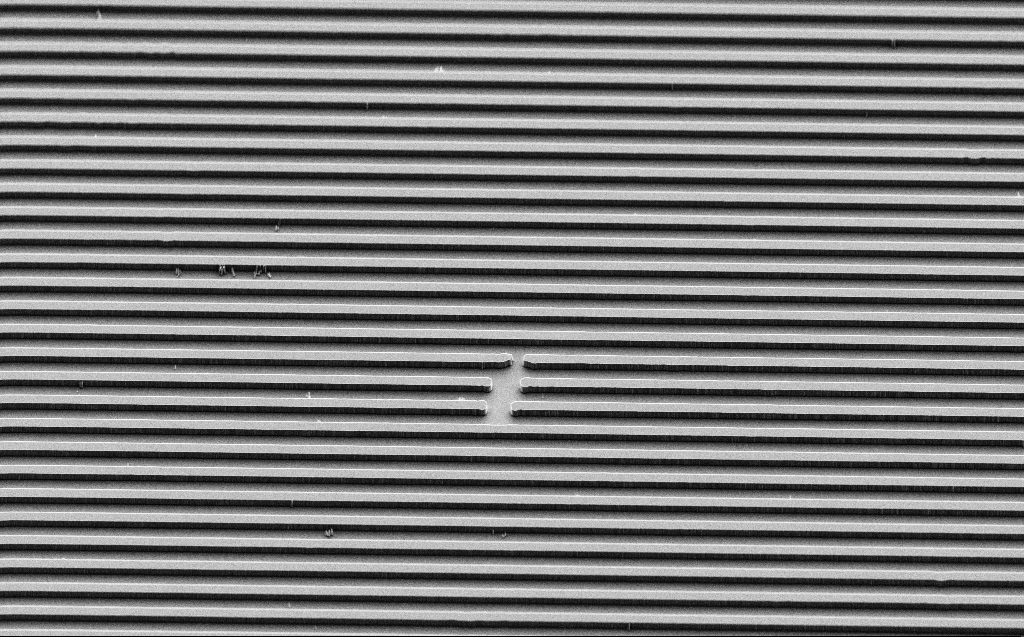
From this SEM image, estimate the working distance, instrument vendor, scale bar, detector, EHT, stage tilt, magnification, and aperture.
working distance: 9 mm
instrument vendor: Zeiss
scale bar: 10000 nm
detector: SE2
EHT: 3 kV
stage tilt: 45°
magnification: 2.19 K X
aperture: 30 µm